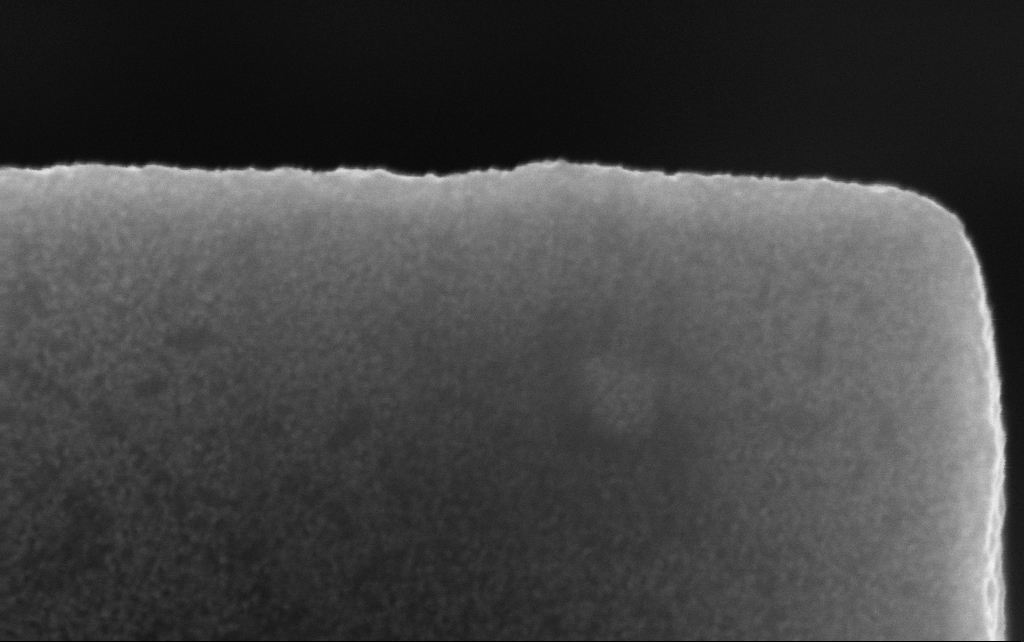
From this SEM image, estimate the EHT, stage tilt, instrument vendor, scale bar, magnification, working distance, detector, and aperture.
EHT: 10 kV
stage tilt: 0°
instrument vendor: Zeiss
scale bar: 200 nm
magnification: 262.28 K X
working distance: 2.5 mm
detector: InLens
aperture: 30 µm